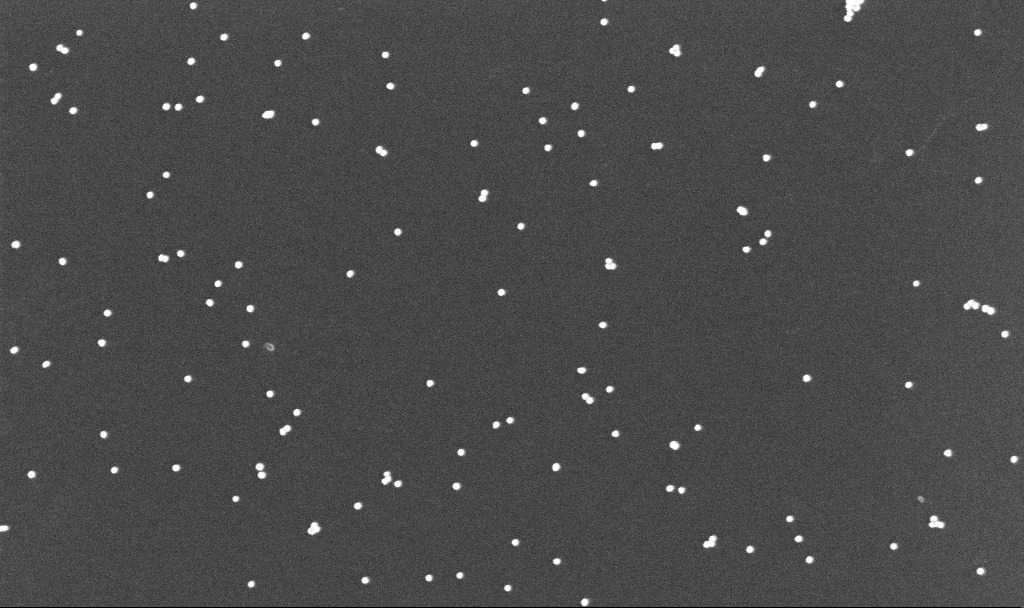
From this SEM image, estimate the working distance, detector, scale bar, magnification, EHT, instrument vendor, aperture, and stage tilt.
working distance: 3.4 mm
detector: InLens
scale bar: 200 nm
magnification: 100 K X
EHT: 10 kV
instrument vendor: Zeiss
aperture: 30 µm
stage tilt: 0°